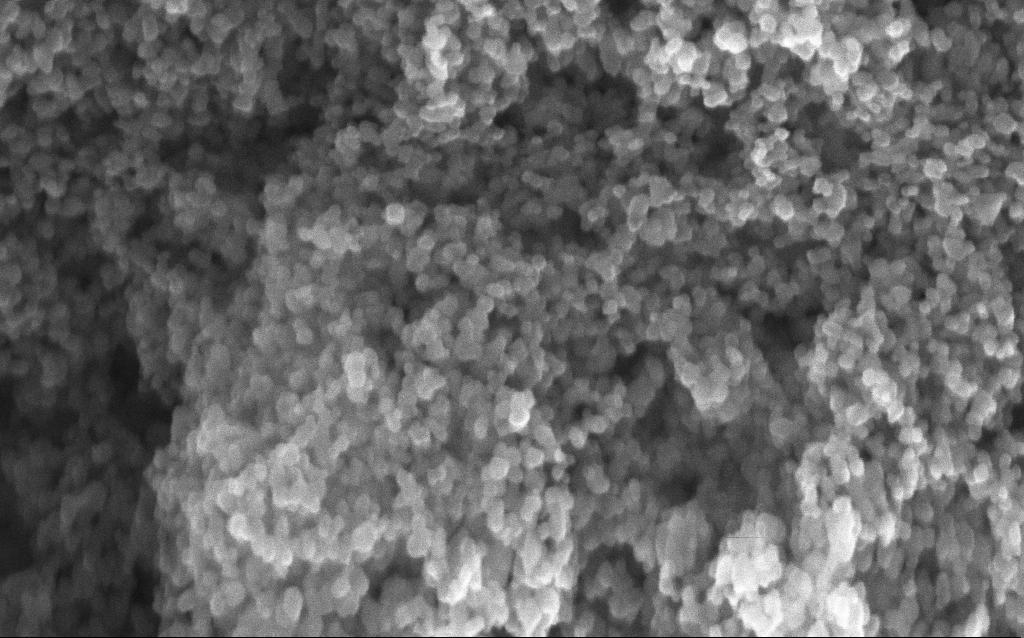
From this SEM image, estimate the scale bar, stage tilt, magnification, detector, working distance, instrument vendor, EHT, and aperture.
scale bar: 100 nm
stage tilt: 0°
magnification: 234.1 K X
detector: InLens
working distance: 2.8 mm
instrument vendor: Zeiss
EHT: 10 kV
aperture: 30 µm